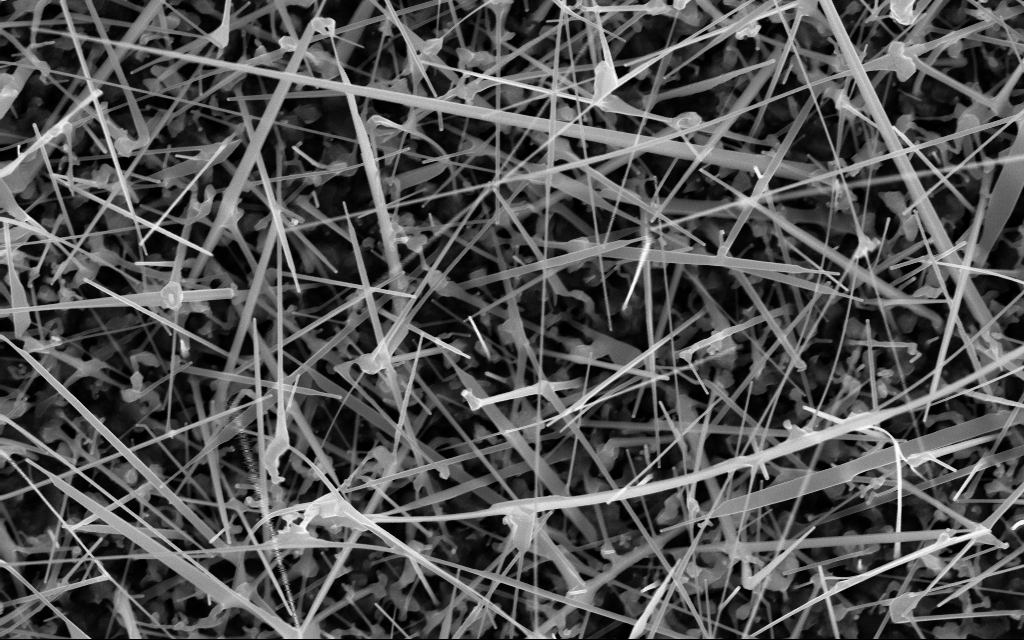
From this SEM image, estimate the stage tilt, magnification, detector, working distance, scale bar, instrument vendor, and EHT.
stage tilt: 0°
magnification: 40 K X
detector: InLens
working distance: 7 mm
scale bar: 1000 nm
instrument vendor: Zeiss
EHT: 10 kV